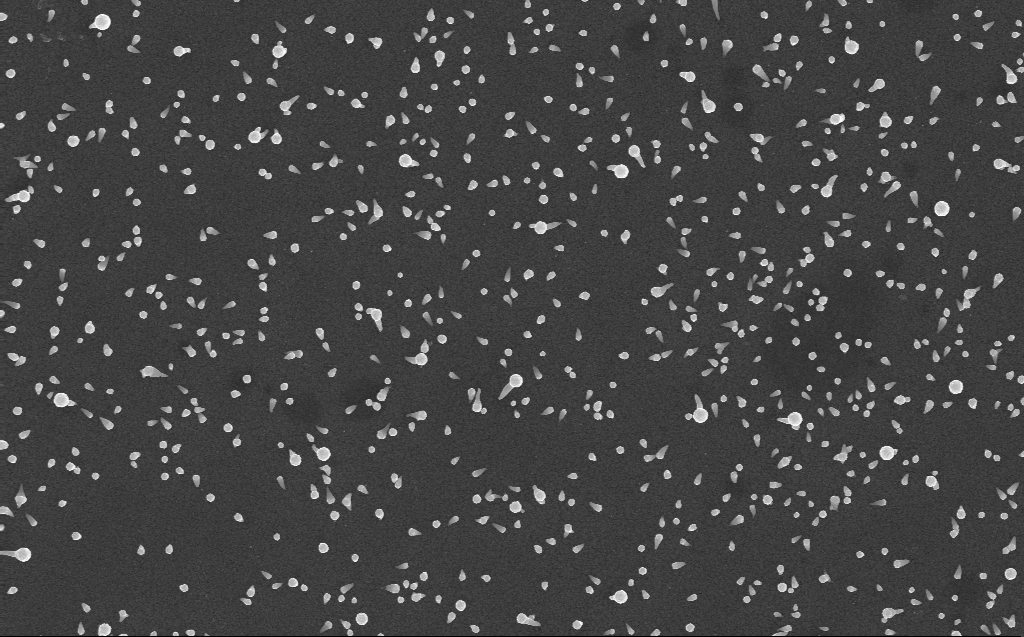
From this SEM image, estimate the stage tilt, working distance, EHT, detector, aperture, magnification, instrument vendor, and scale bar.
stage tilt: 0°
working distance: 3 mm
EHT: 10 kV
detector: InLens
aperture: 30 µm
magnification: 18.77 K X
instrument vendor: Zeiss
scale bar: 1000 nm